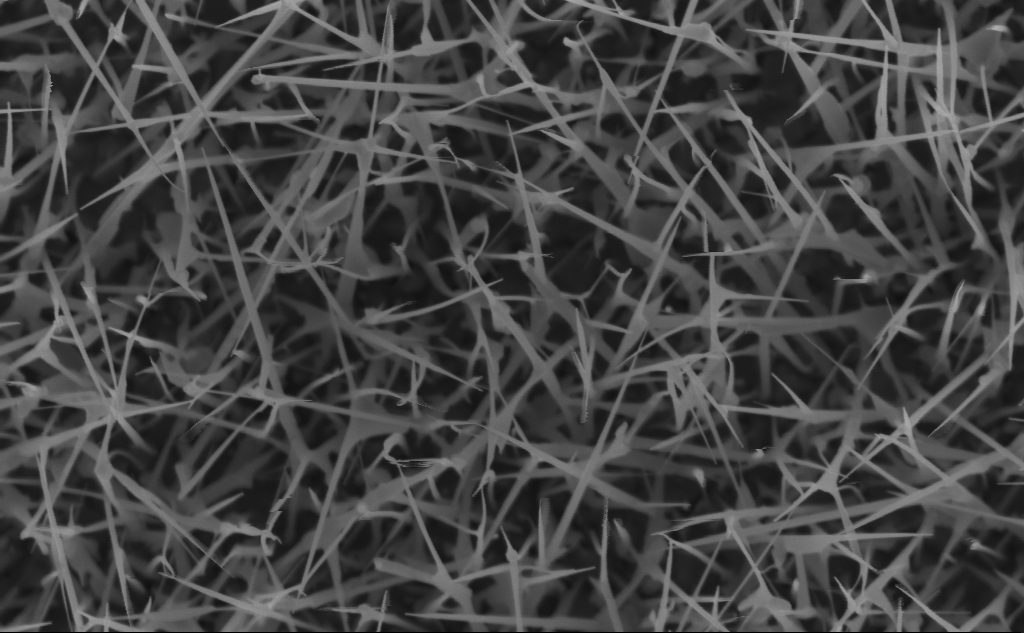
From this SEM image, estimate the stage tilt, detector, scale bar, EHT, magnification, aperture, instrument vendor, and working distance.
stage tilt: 0°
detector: InLens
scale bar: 1000 nm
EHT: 5 kV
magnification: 47.71 K X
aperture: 30 µm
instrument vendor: Zeiss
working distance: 6 mm